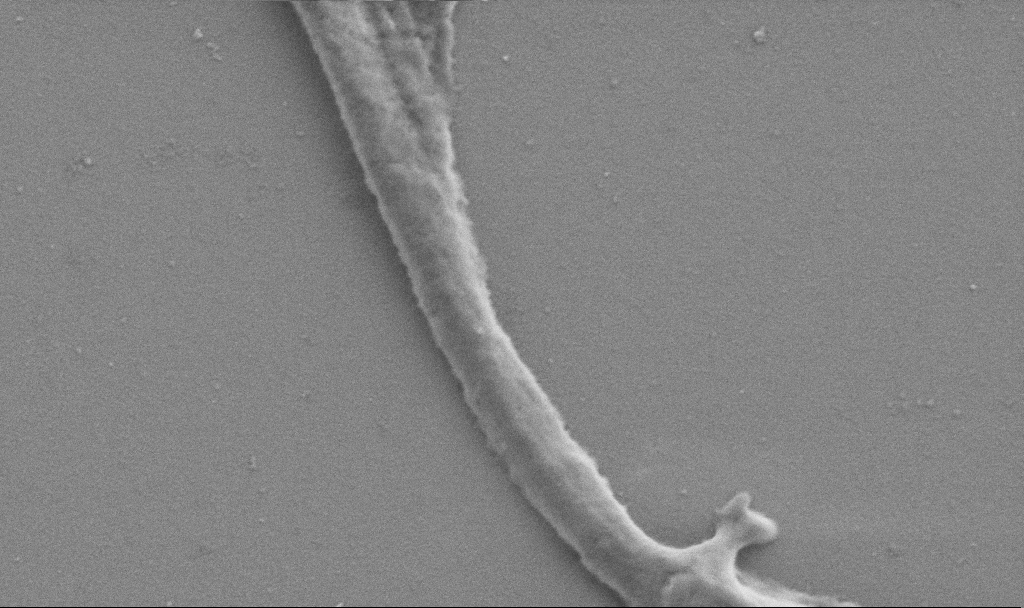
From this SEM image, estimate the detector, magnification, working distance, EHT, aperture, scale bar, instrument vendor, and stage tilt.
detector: SE2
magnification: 50 K X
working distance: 6.9 mm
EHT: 0.9 kV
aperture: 30 µm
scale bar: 1000 nm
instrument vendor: Zeiss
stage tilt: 0°